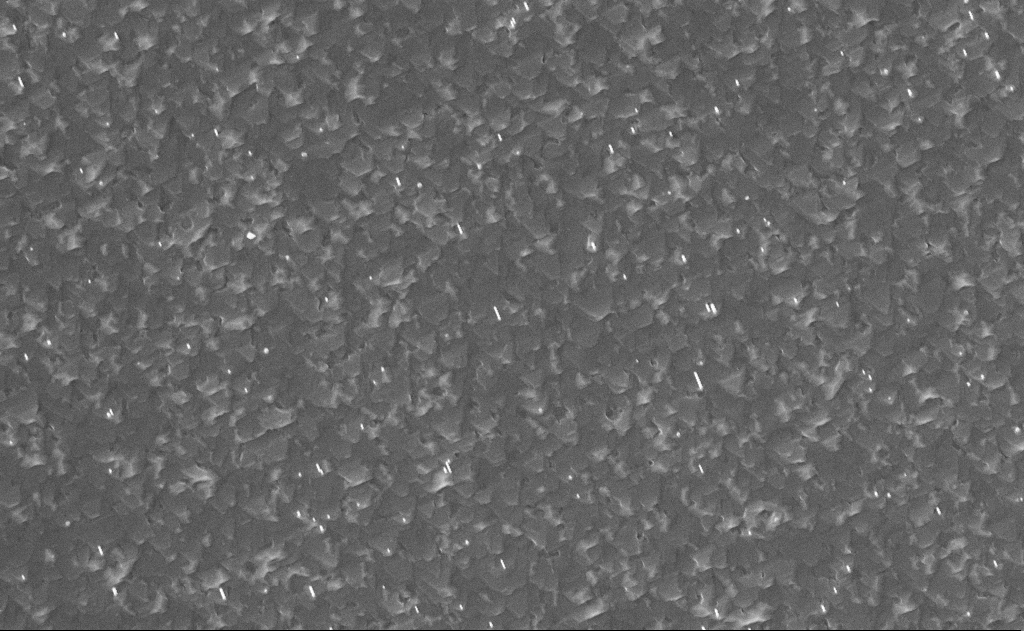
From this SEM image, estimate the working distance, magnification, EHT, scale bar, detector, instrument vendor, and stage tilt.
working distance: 14 mm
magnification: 20 K X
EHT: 10 kV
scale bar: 1000 nm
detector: InLens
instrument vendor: Zeiss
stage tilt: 0°